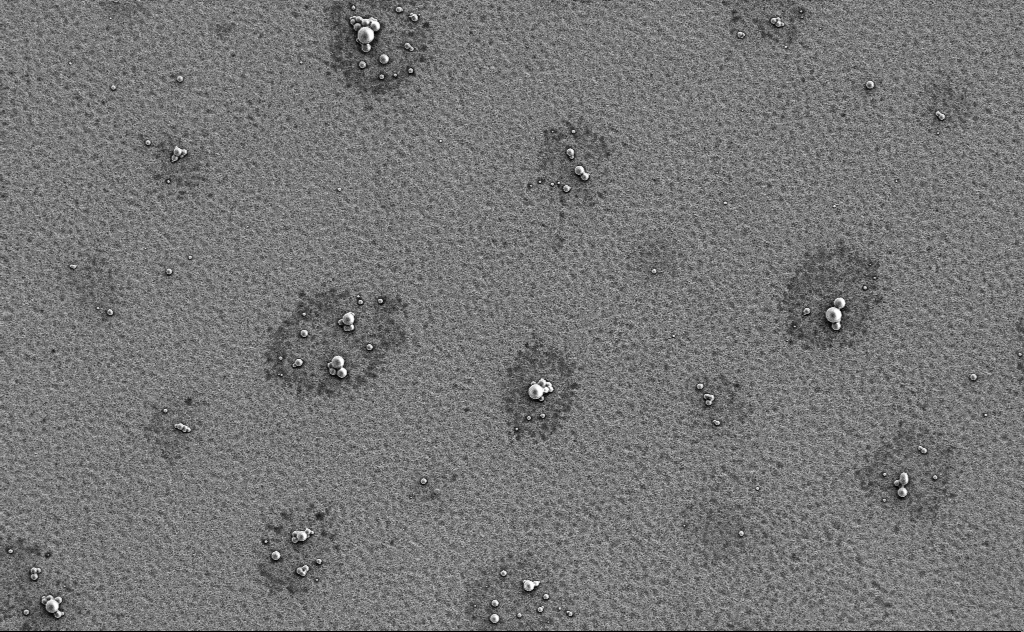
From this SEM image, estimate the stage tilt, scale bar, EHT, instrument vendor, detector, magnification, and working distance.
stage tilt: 0°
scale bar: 10000 nm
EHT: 3 kV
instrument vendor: Zeiss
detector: SE2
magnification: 2.66 K X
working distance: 13 mm